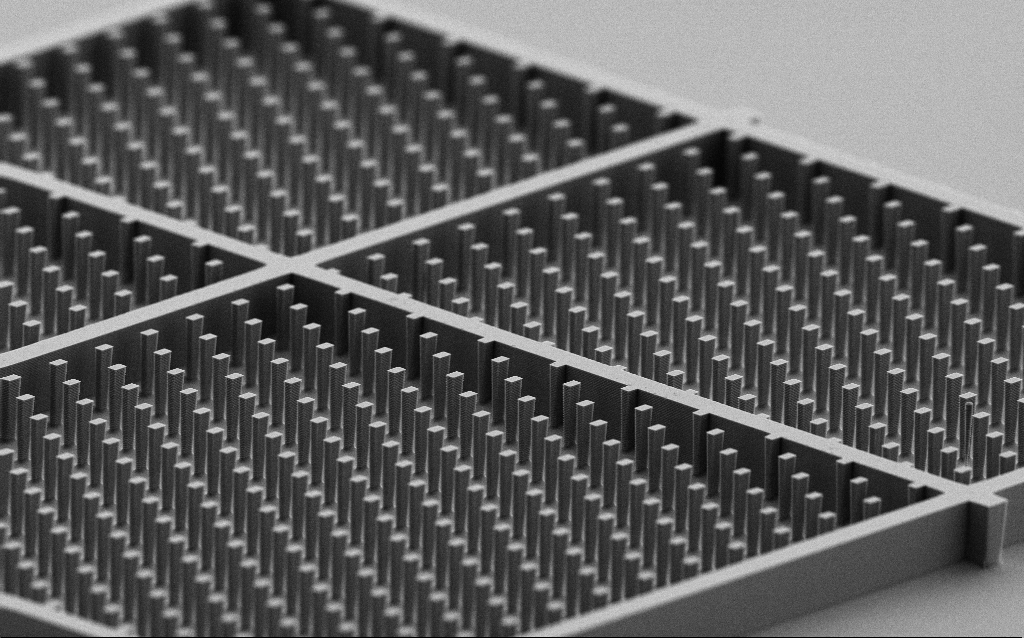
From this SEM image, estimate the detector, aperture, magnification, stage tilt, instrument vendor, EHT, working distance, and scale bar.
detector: SE2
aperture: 30 µm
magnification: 2.03 K X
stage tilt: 70°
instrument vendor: Zeiss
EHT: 6 kV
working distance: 5.9 mm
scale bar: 10000 nm